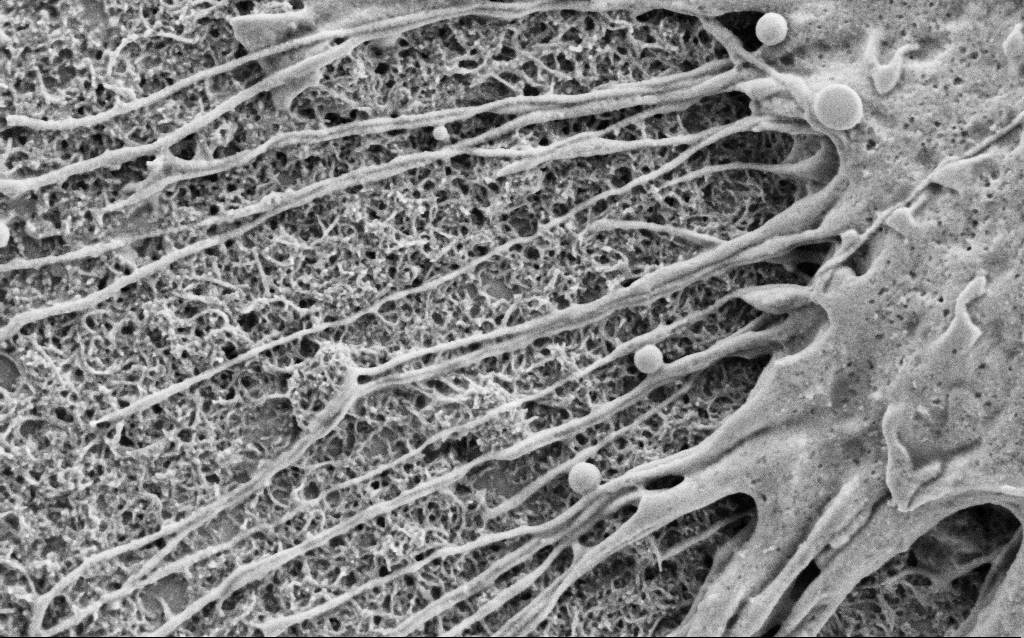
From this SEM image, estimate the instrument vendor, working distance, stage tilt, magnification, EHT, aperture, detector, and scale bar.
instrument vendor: Zeiss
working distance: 6.8 mm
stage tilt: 0°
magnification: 25 K X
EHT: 2 kV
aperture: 30 µm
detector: SE2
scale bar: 1000 nm